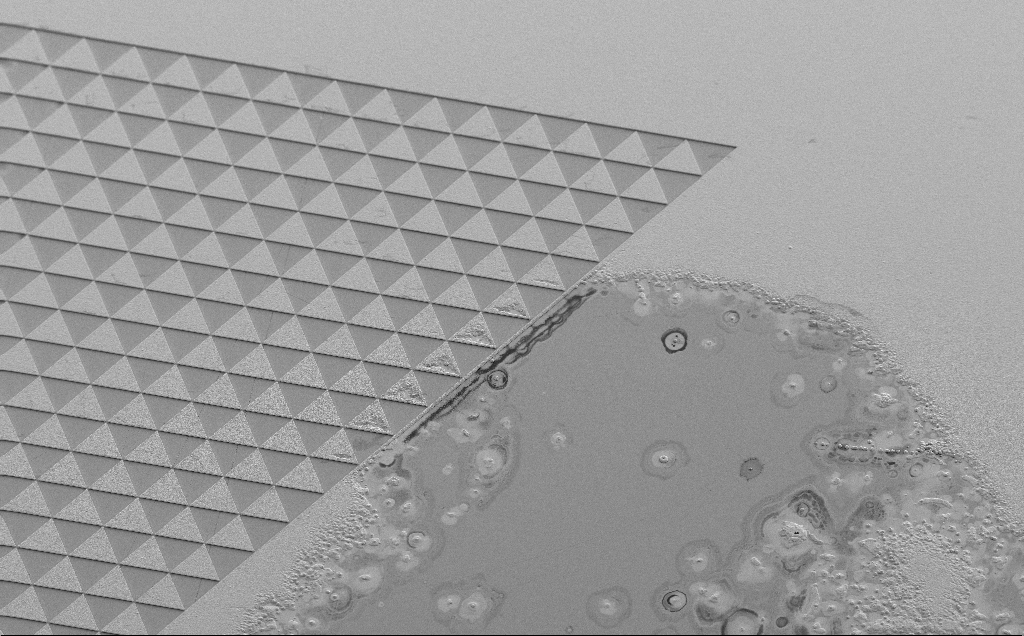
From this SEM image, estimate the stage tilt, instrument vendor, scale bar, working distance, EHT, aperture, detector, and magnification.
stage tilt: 35°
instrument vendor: Zeiss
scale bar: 100000 nm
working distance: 13 mm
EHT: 5 kV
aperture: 30 µm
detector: SE2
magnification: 0.316 K X